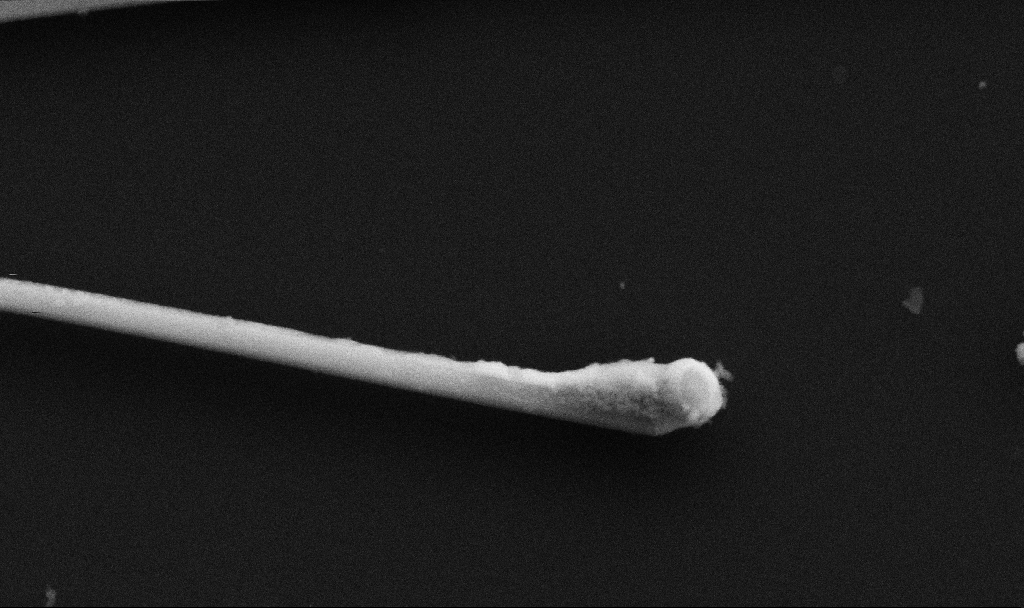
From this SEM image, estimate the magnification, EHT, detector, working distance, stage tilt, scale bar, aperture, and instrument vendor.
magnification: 118.35 K X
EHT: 5 kV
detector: SE2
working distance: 6.7 mm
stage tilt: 0°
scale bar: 200 nm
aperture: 30 µm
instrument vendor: Zeiss